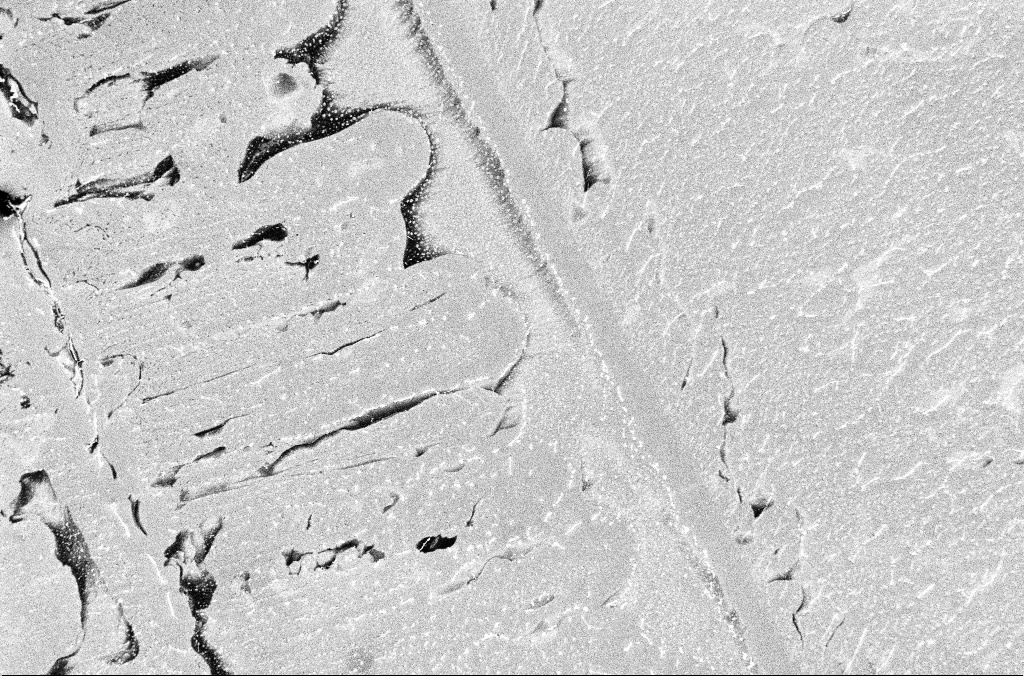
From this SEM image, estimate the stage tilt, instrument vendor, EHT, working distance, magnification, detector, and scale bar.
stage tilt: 0°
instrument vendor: Zeiss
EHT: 3 kV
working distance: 4.5 mm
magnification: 0.545 K X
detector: InLens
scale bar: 100000 nm